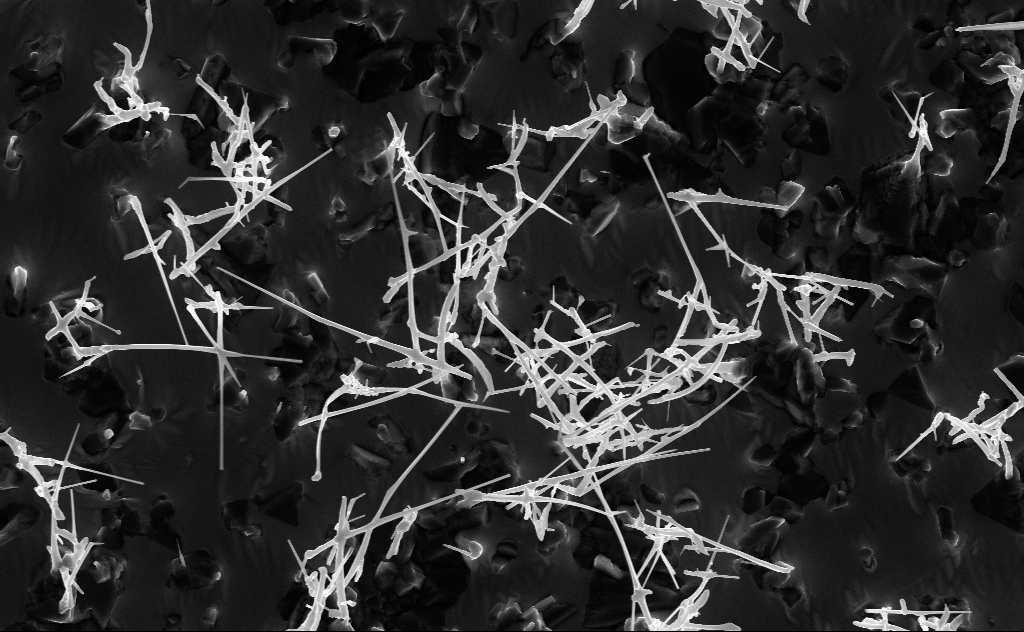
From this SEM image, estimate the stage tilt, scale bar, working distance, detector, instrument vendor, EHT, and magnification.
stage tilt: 0°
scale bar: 2000 nm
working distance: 6 mm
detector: InLens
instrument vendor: Zeiss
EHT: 10 kV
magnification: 10 K X